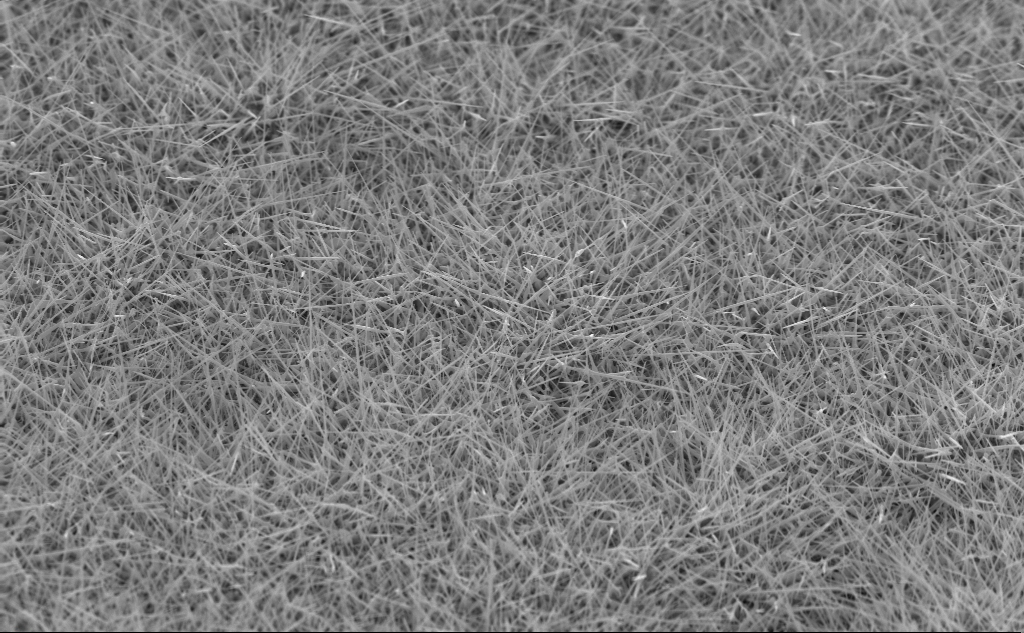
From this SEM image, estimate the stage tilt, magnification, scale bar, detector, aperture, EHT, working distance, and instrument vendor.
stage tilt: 45°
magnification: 10 K X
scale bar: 2000 nm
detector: InLens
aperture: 30 µm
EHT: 10 kV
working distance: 4 mm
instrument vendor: Zeiss